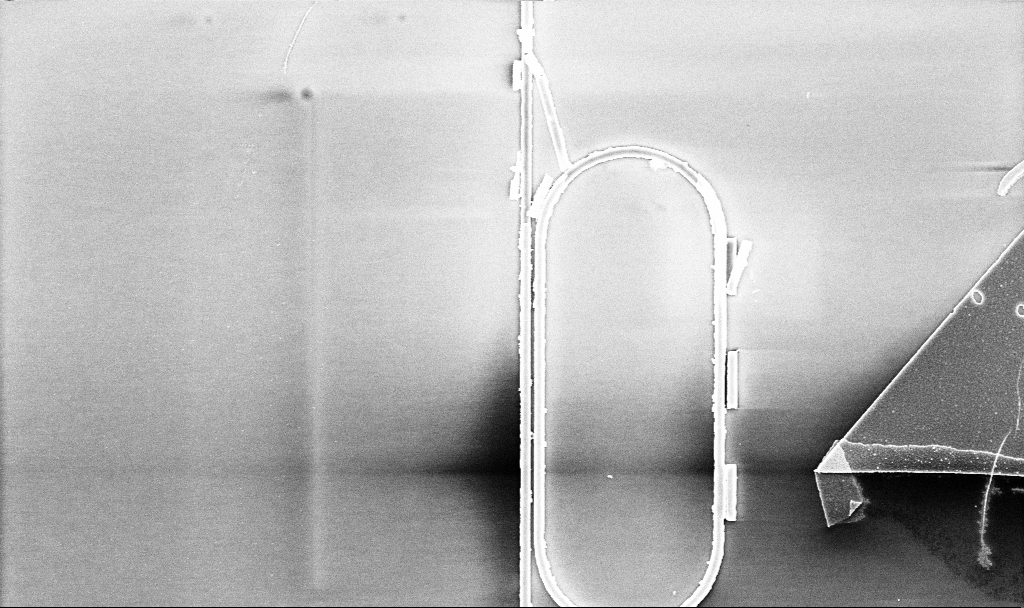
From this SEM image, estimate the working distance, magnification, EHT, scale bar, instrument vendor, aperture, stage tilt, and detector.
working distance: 7.5 mm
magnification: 6.98 K X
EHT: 5 kV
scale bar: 10000 nm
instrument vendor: Zeiss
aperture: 30 µm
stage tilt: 0°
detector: InLens